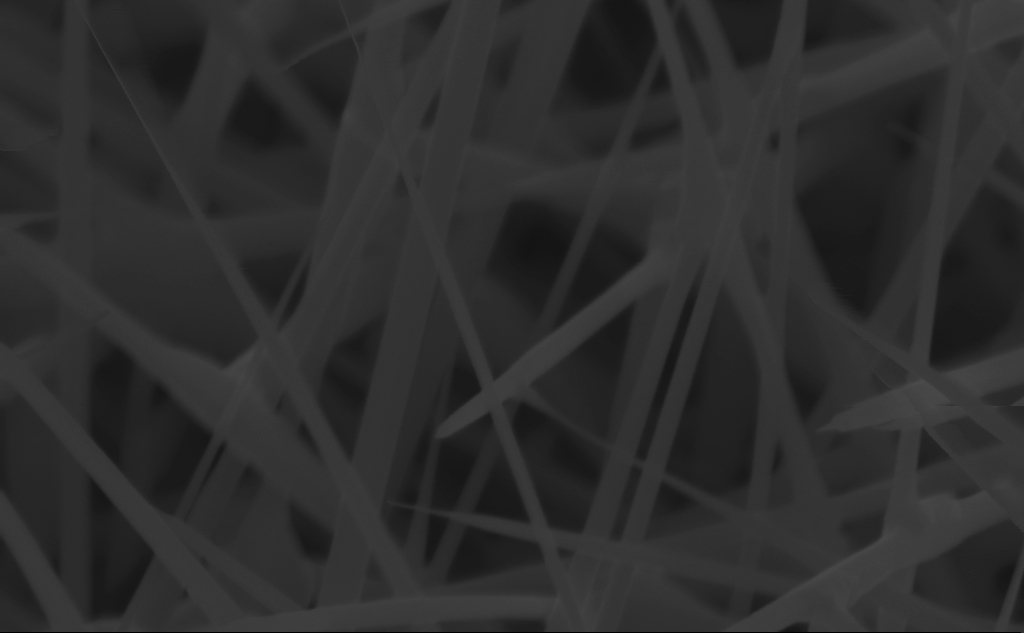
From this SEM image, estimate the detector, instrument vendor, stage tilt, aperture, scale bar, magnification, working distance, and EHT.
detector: InLens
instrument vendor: Zeiss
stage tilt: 45°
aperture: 30 µm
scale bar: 200 nm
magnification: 150 K X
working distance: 5 mm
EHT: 10 kV